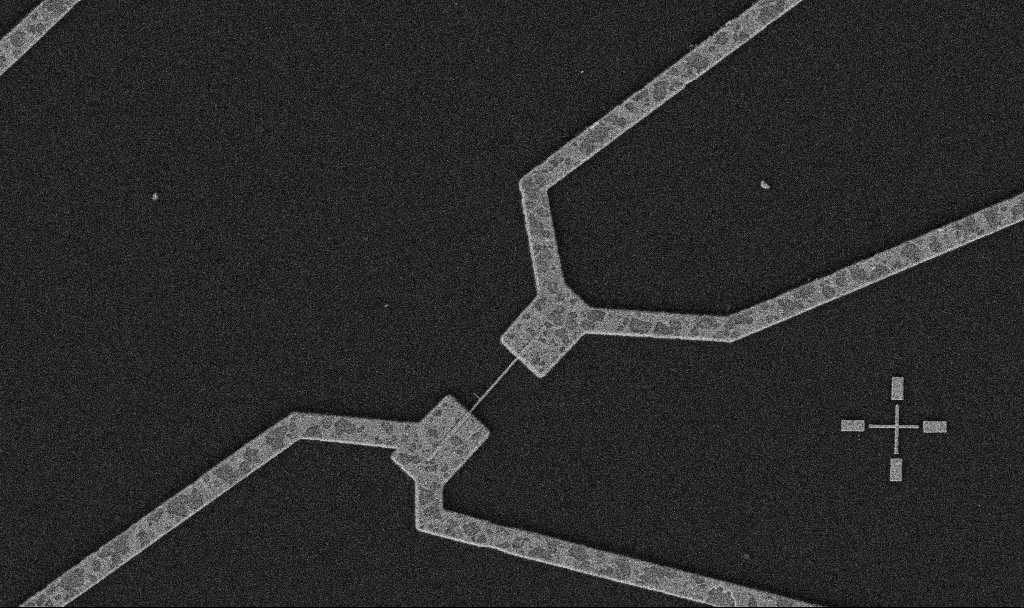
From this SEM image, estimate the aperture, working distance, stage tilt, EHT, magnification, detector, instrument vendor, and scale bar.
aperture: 30 µm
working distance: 10.7 mm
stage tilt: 0°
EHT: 5 kV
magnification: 10 K X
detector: SE2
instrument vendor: Zeiss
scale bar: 2000 nm